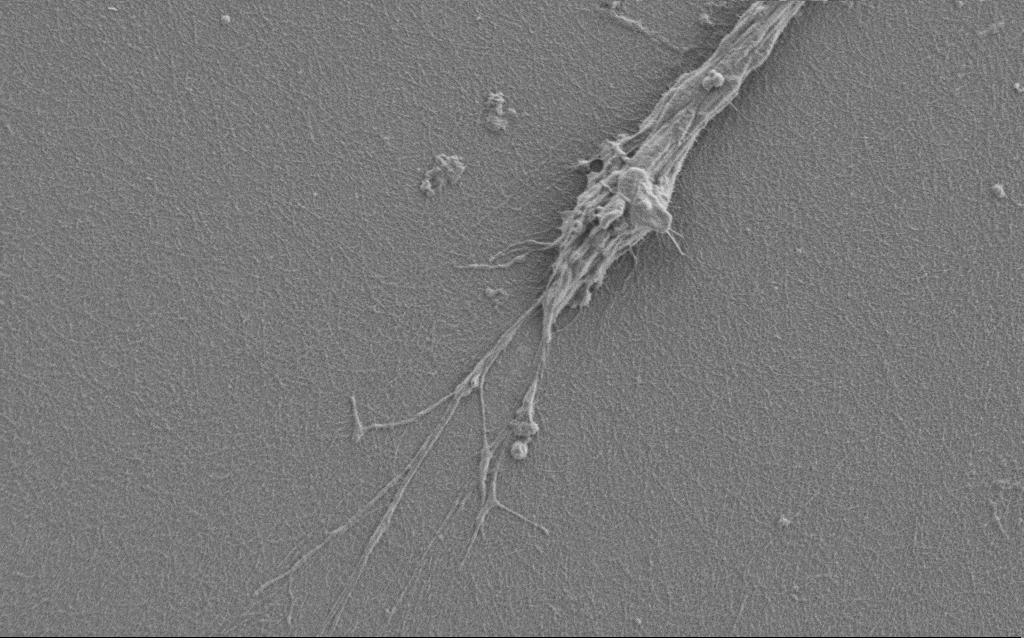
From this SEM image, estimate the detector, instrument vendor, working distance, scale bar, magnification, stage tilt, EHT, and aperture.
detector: SE2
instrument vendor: Zeiss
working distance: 6 mm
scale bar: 2000 nm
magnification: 7.5 K X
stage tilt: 0°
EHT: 1 kV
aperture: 30 µm